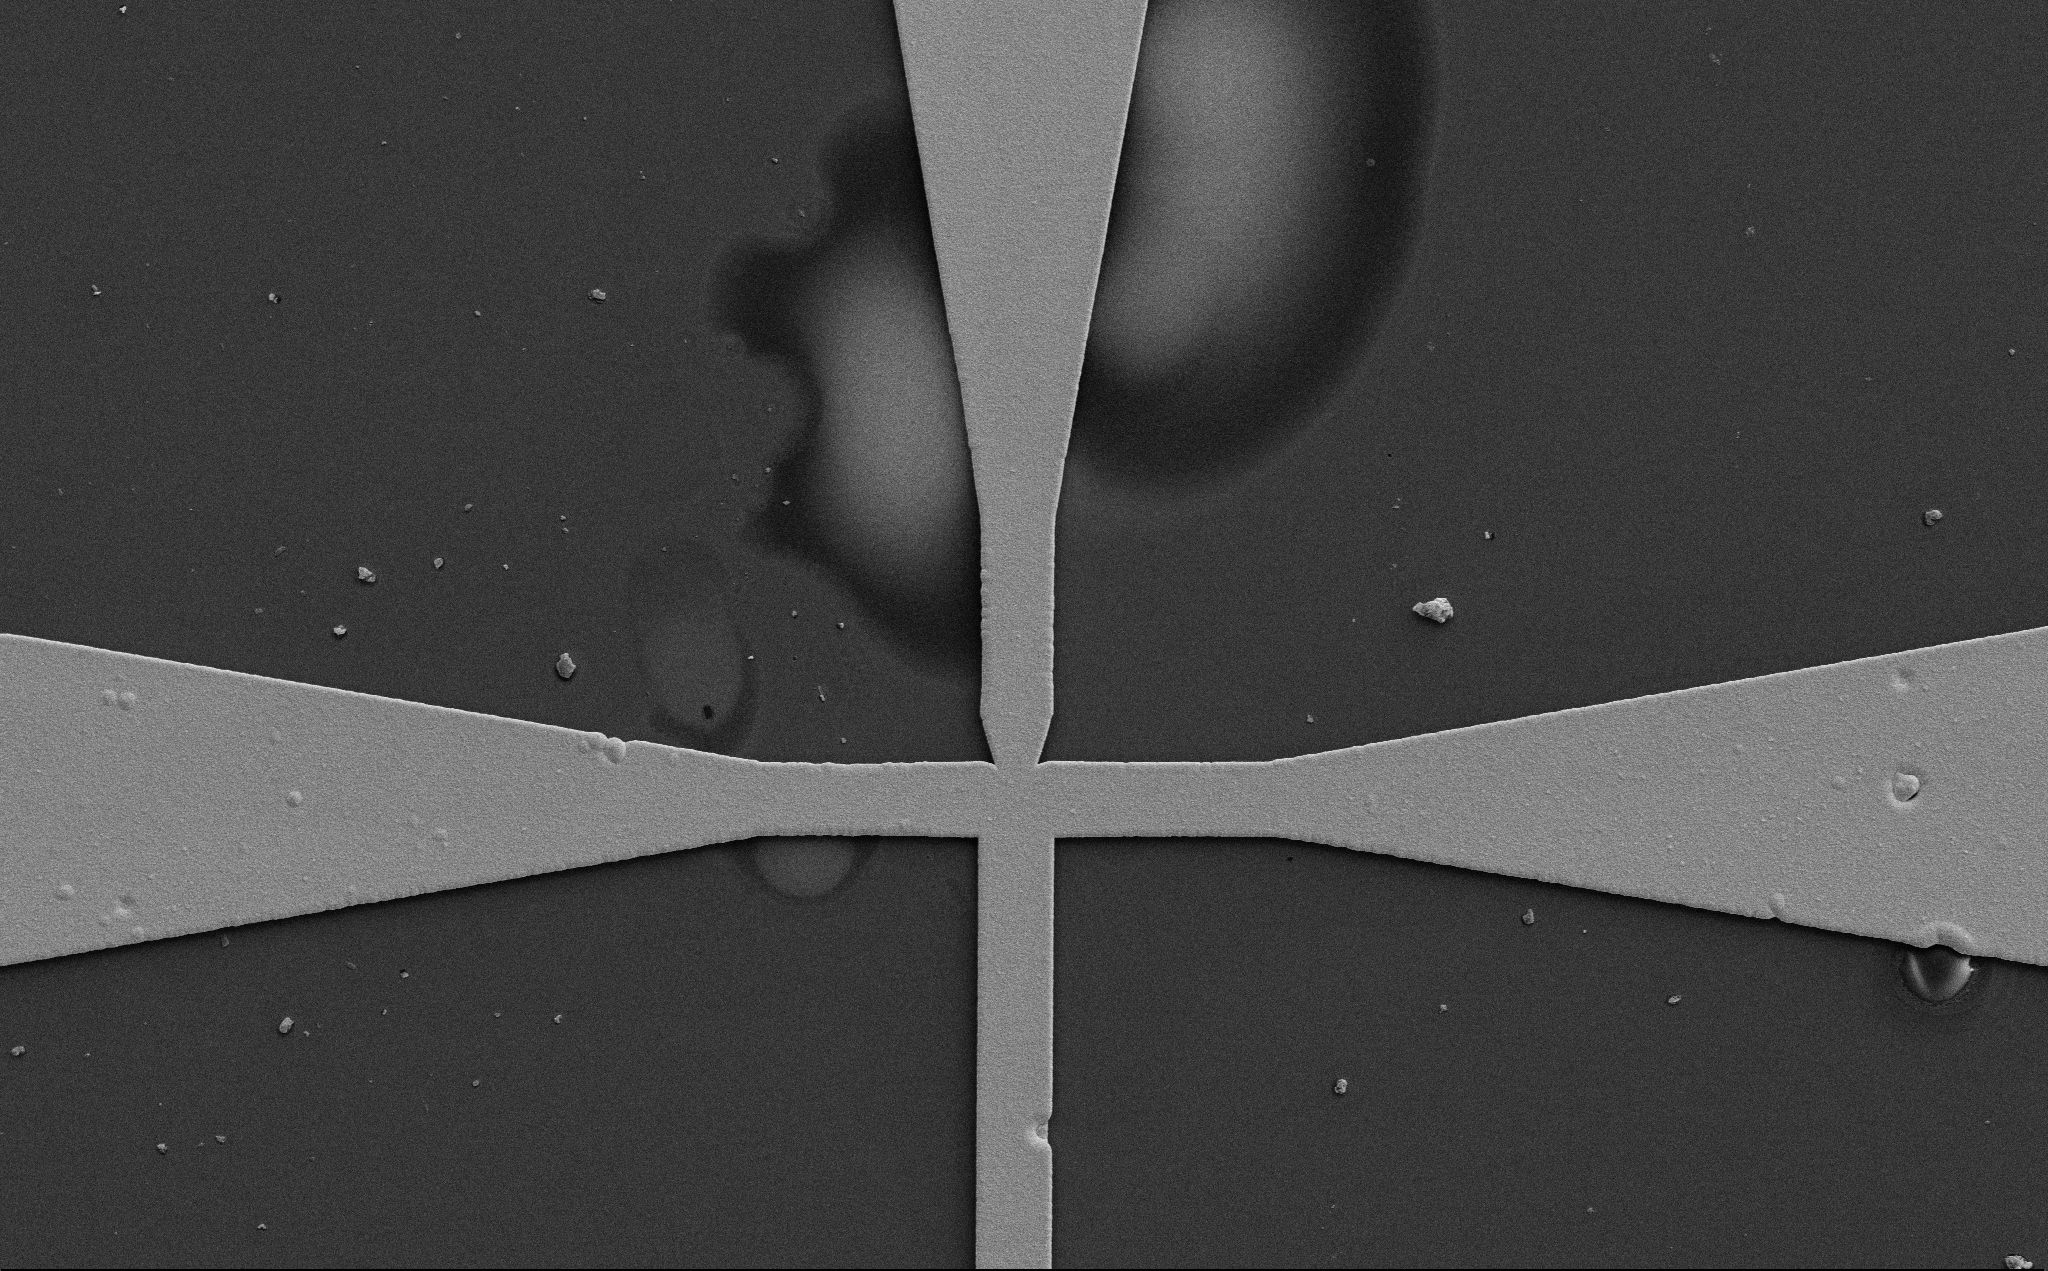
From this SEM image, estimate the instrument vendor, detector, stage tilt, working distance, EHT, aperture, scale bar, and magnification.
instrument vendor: Zeiss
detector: SE2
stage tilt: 0°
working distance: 12 mm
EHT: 10 kV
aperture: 30 µm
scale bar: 20000 nm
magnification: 1.21 K X